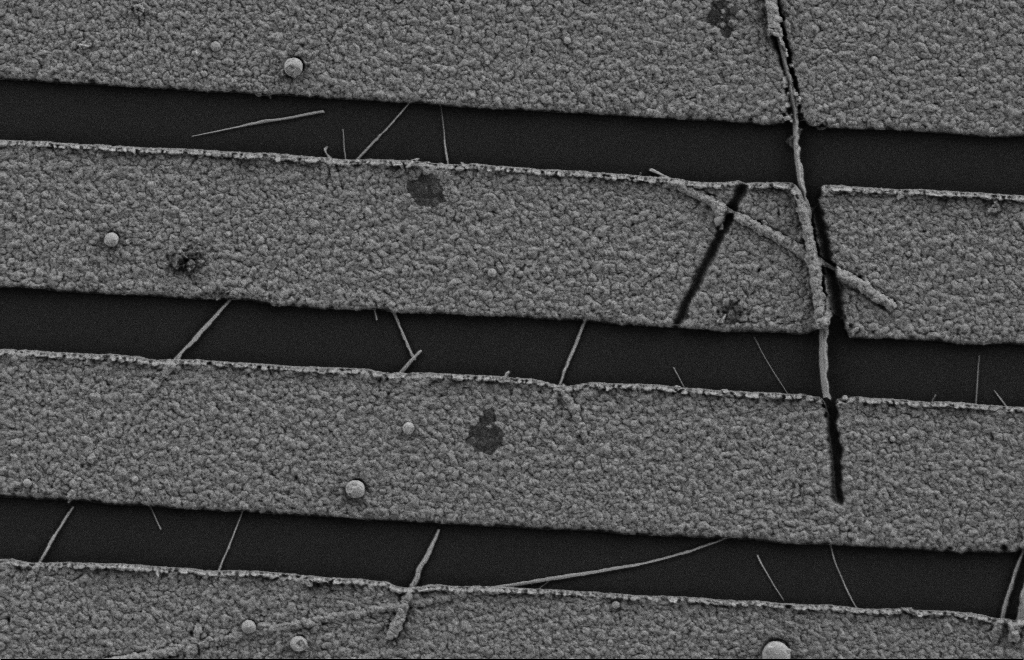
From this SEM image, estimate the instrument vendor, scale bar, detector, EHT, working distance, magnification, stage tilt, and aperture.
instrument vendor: Zeiss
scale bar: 2000 nm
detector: SE2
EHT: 2 kV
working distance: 10 mm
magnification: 19.06 K X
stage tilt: -0.3°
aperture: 20 µm